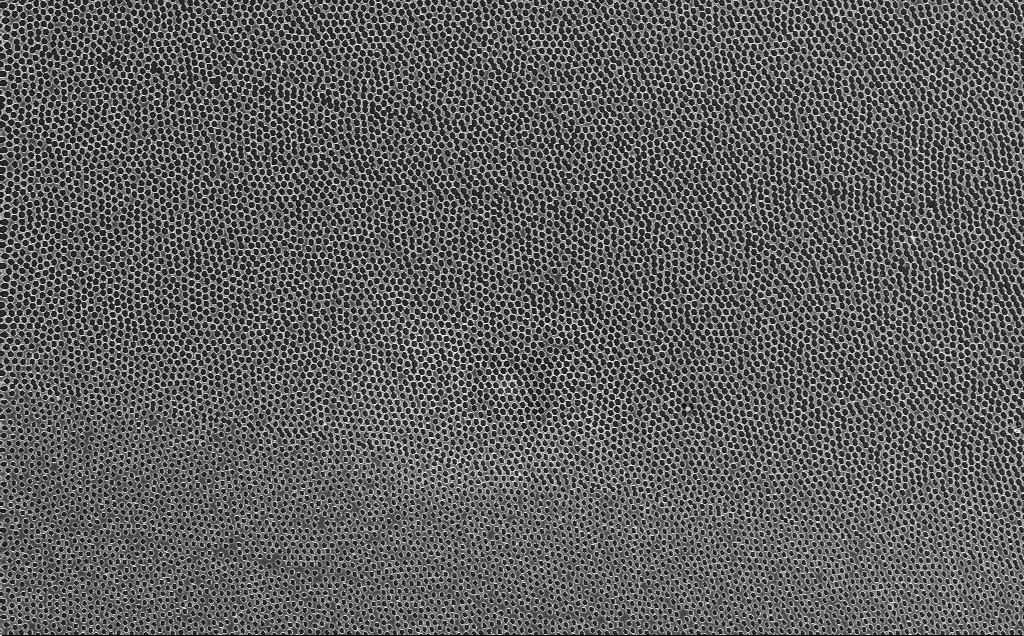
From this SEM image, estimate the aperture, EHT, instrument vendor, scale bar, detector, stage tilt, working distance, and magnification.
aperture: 30 µm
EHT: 3 kV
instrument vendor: Zeiss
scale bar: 2000 nm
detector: InLens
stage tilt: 0°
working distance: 2.4 mm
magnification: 8.21 K X